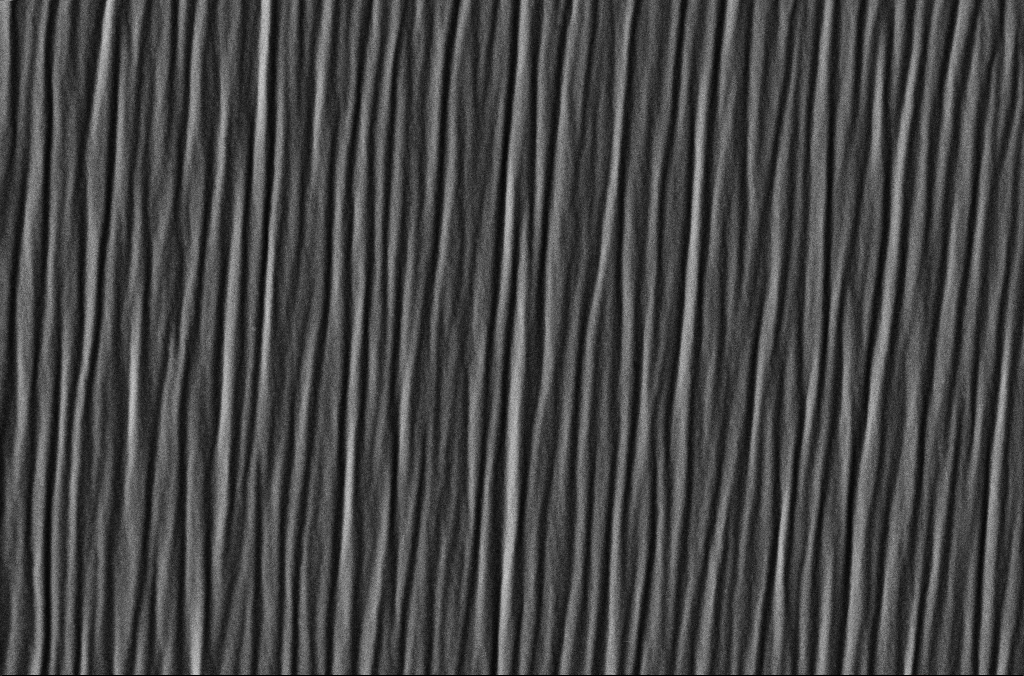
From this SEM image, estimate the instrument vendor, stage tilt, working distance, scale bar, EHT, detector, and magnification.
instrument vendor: Zeiss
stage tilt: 0°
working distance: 5 mm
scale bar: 2000 nm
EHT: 3 kV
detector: SE2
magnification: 25 K X